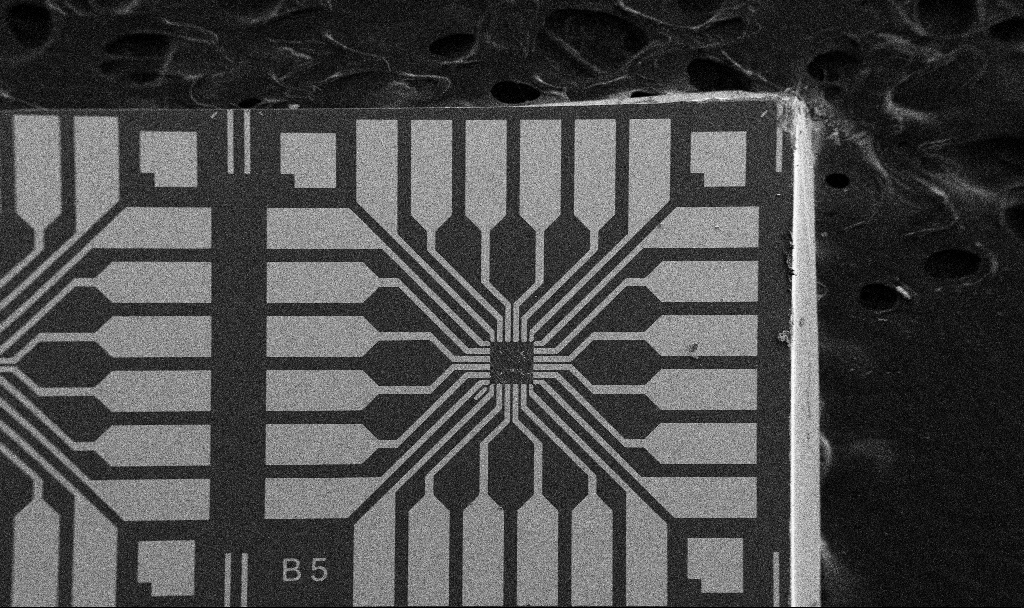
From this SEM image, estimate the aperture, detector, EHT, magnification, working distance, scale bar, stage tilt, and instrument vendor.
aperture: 30 µm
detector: SE2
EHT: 5 kV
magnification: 0.1 K X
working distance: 10.7 mm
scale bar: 200000 nm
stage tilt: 0°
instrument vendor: Zeiss